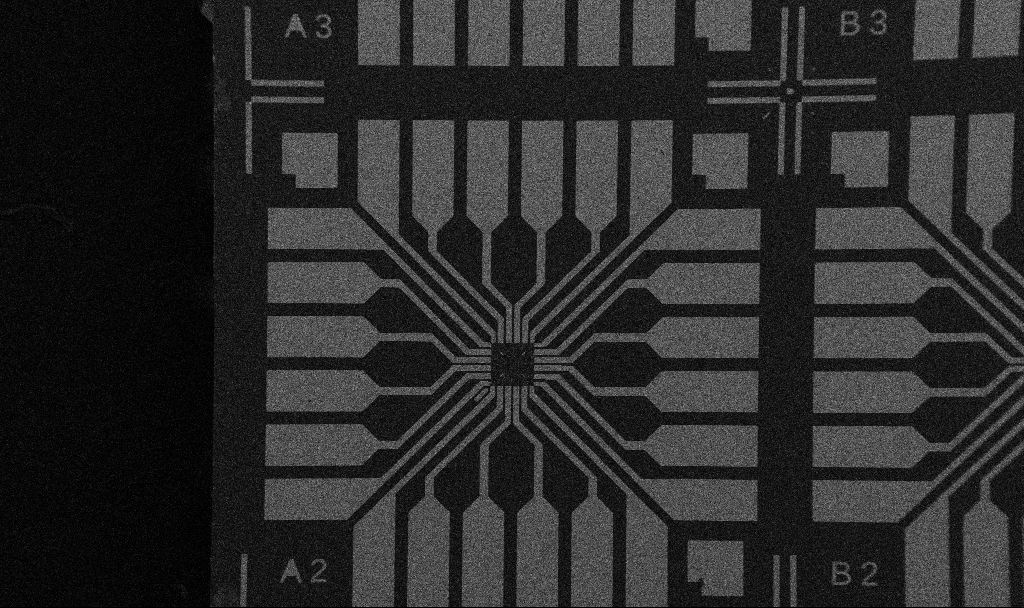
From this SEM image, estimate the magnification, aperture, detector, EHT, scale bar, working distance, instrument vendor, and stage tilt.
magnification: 0.1 K X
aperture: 30 µm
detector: SE2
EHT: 5 kV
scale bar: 200000 nm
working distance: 10.7 mm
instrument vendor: Zeiss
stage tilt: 0°